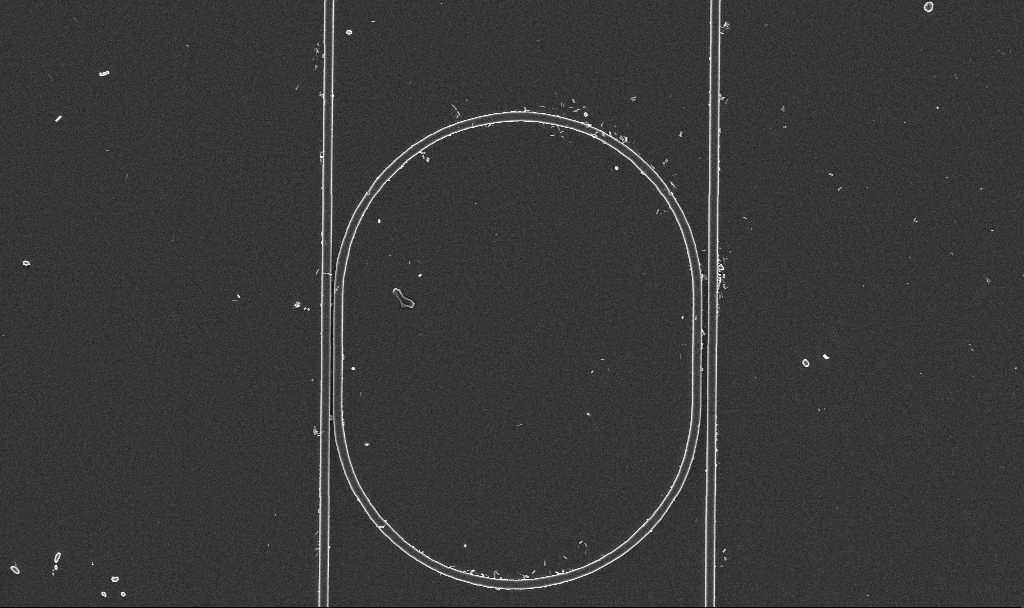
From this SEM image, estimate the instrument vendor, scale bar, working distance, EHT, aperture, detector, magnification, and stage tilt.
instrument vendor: Zeiss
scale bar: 10000 nm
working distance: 2.9 mm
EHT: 3 kV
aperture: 30 µm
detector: InLens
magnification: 6.85 K X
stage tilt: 0°